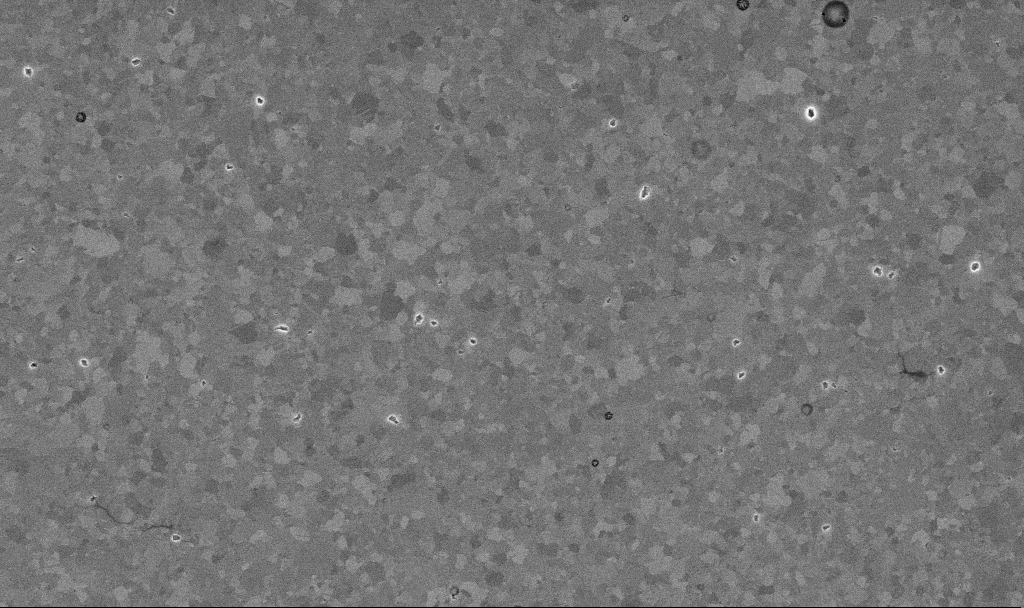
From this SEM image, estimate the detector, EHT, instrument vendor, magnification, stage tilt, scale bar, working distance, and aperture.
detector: InLens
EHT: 10 kV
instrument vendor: Zeiss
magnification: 20.87 K X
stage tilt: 0°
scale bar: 1000 nm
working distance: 3.3 mm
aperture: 30 µm